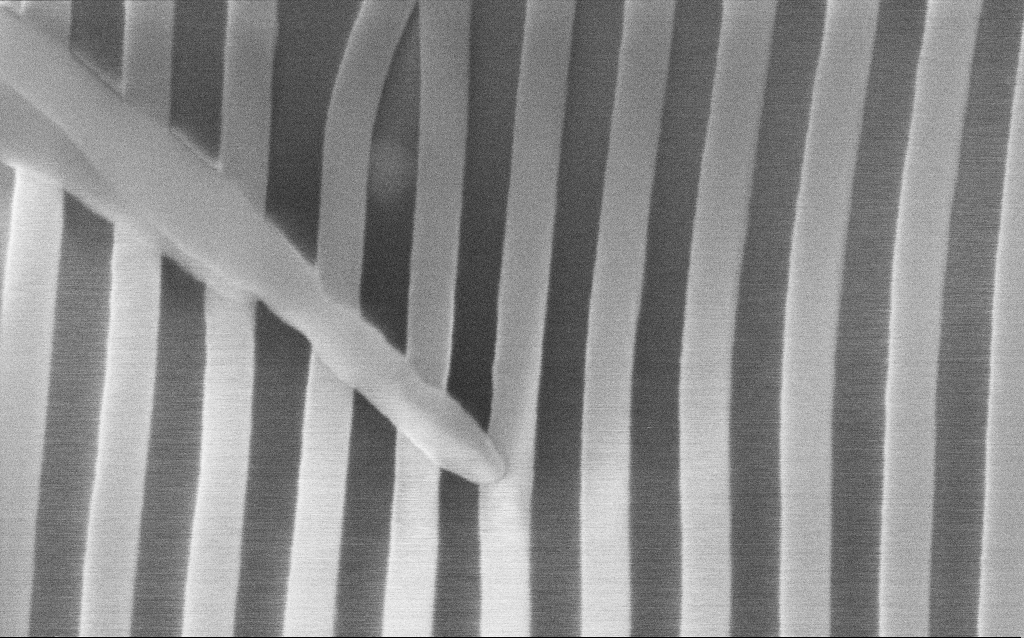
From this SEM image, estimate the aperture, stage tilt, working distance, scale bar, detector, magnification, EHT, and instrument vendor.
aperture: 30 µm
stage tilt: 45°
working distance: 7.9 mm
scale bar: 200 nm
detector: InLens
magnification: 215.86 K X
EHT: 5 kV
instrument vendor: Zeiss